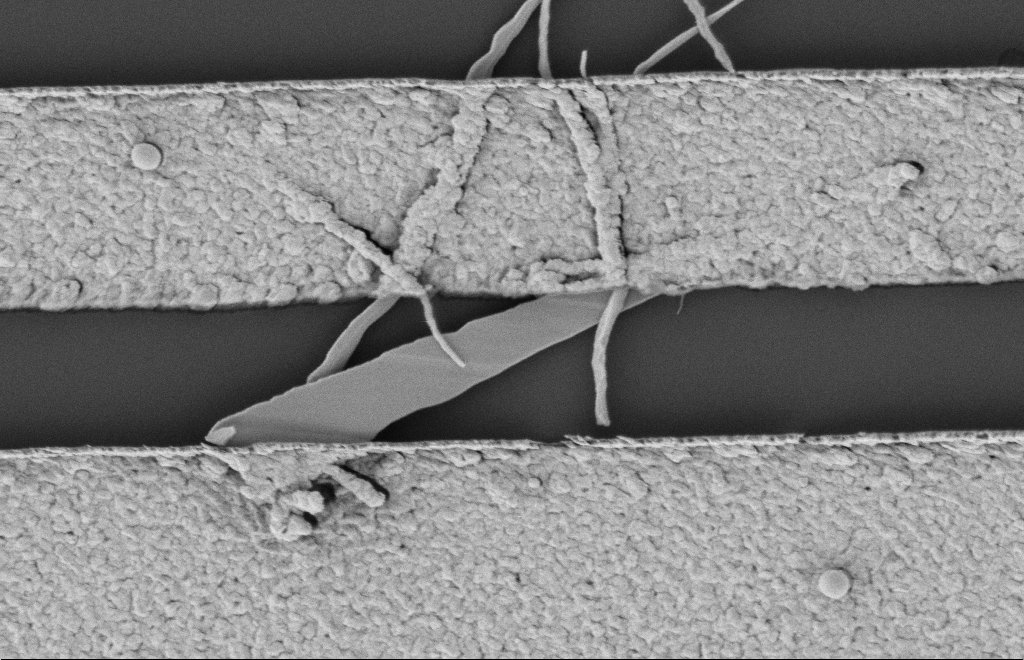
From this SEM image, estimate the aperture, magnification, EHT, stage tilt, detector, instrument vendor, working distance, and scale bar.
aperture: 20 µm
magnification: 33.11 K X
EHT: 2 kV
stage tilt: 0°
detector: SE2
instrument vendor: Zeiss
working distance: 12 mm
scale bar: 1000 nm